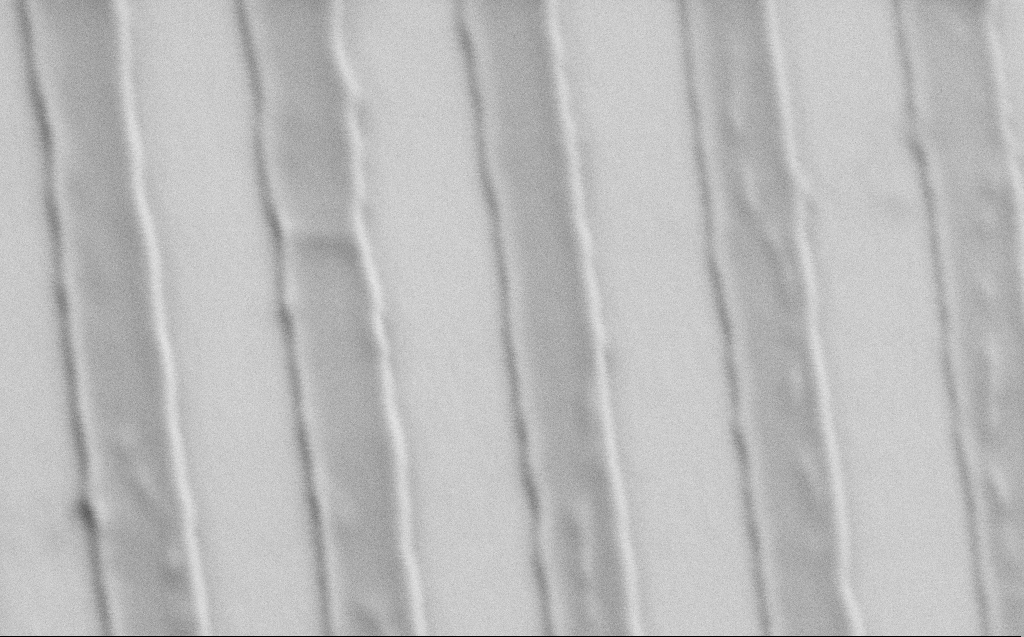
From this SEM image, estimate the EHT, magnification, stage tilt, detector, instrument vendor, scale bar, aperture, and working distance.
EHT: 1 kV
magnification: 162.41 K X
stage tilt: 60.7°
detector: SE2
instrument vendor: Zeiss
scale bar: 200 nm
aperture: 30 µm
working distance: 3 mm